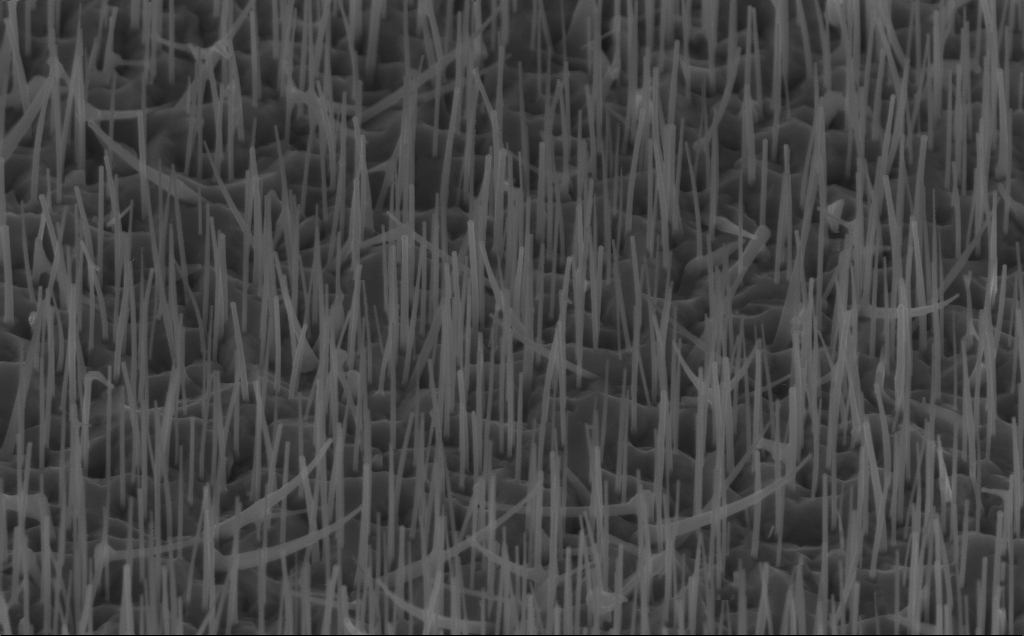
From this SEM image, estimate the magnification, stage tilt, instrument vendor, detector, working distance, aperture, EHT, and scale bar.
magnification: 40 K X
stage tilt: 45°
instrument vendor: Zeiss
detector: InLens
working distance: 5 mm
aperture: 30 µm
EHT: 10 kV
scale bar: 1000 nm